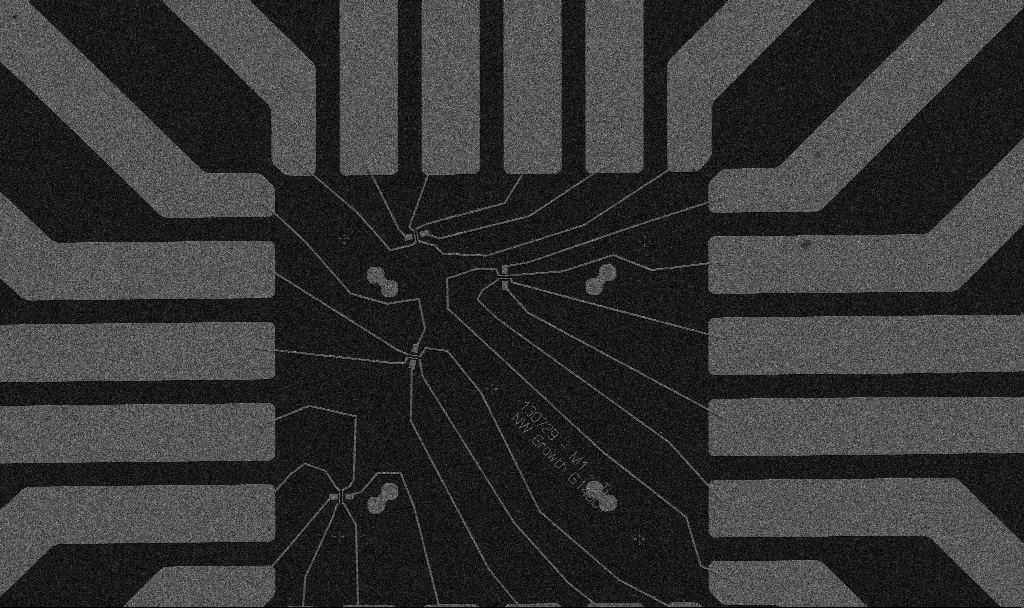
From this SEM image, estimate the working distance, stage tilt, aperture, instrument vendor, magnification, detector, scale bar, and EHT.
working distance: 10.7 mm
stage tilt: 0°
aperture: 30 µm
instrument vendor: Zeiss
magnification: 1 K X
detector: SE2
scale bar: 20000 nm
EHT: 5 kV